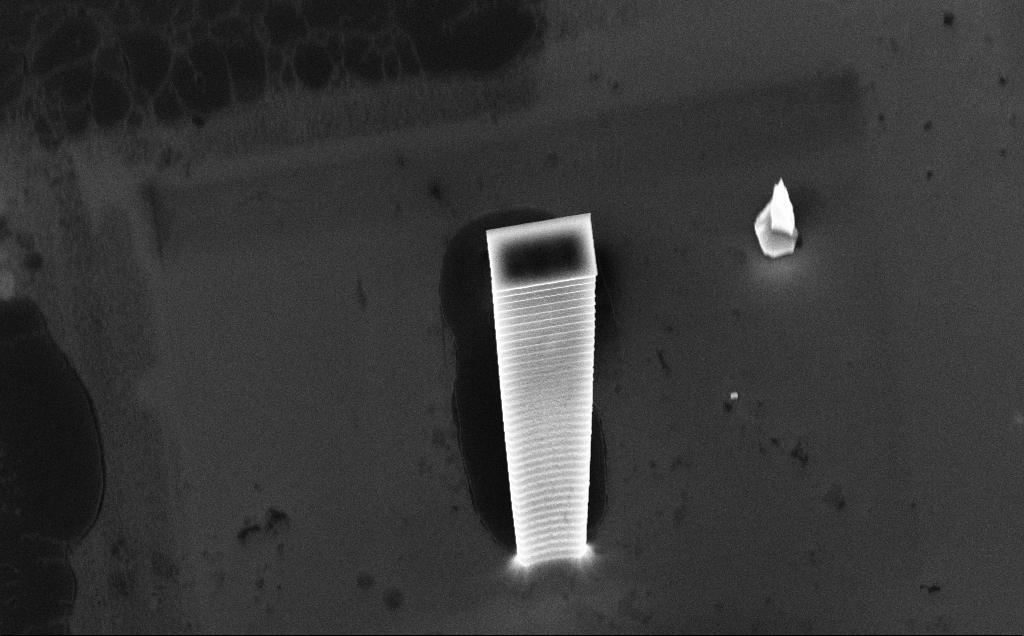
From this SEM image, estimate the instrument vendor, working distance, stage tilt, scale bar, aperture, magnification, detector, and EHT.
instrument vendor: Zeiss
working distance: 5 mm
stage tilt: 45°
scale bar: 2000 nm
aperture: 30 µm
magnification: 8.03 K X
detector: InLens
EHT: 10 kV